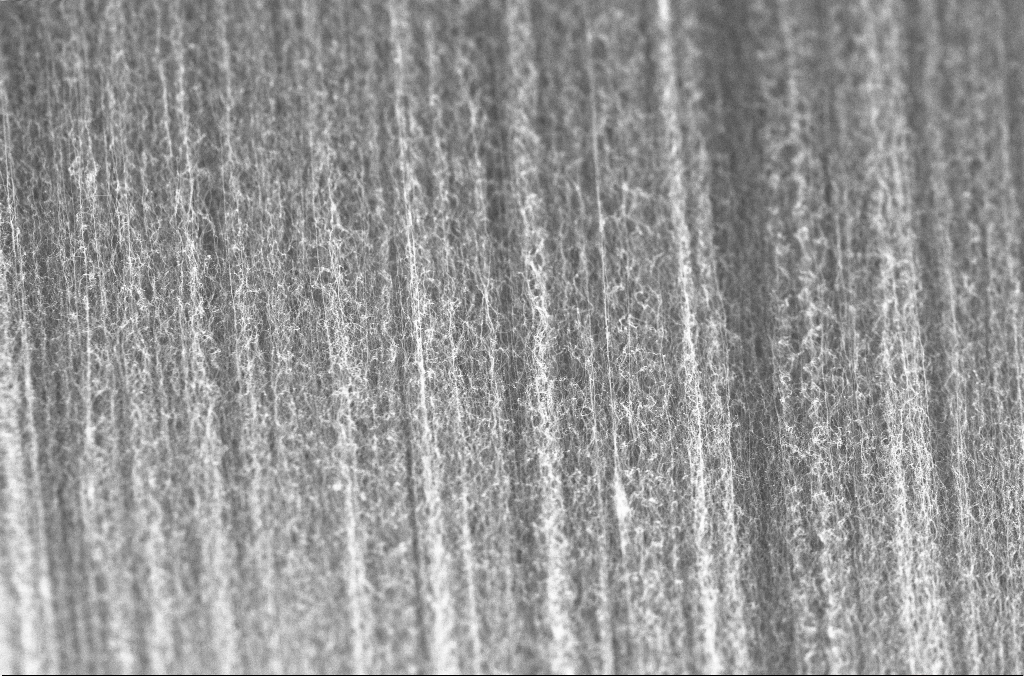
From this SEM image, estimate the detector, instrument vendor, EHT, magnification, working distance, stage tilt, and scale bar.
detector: InLens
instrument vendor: Zeiss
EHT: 20 kV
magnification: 10 K X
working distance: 4 mm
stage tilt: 0°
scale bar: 2000 nm